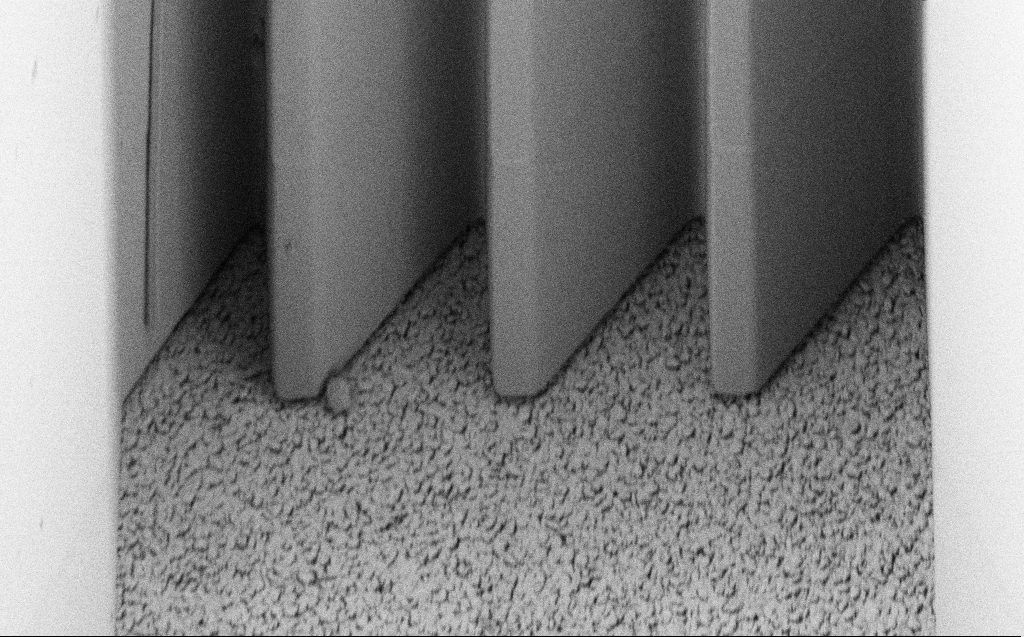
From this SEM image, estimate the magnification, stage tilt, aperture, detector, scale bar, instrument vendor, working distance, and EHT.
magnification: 7 K X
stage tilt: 45°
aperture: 30 µm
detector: SE2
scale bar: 10000 nm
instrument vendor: Zeiss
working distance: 8 mm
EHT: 1 kV